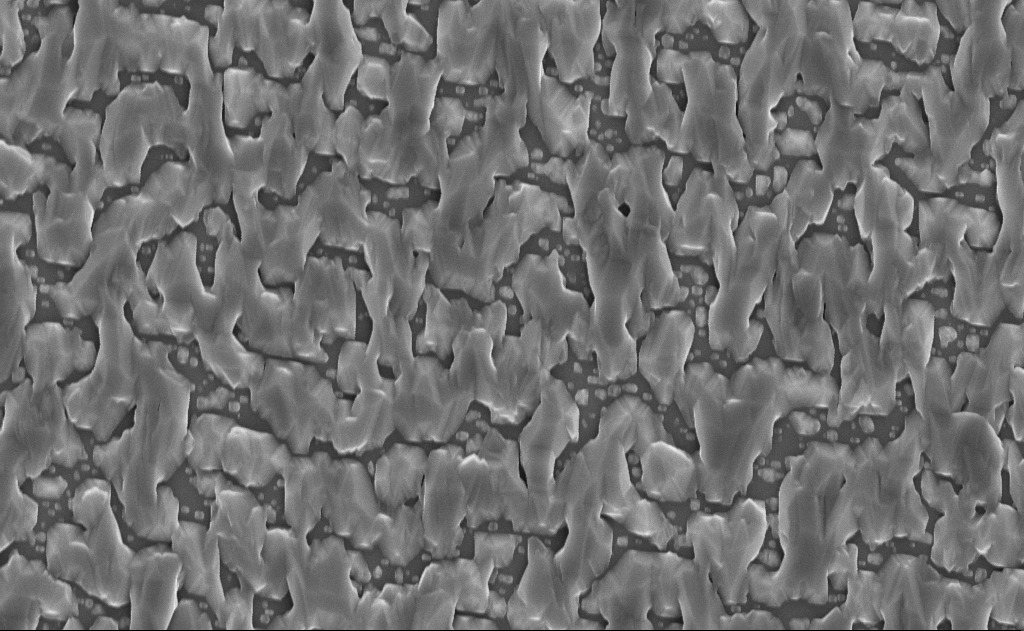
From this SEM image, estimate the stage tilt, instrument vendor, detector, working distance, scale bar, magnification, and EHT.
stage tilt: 0°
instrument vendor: Zeiss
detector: InLens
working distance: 14 mm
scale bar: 2000 nm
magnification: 20 K X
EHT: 10 kV